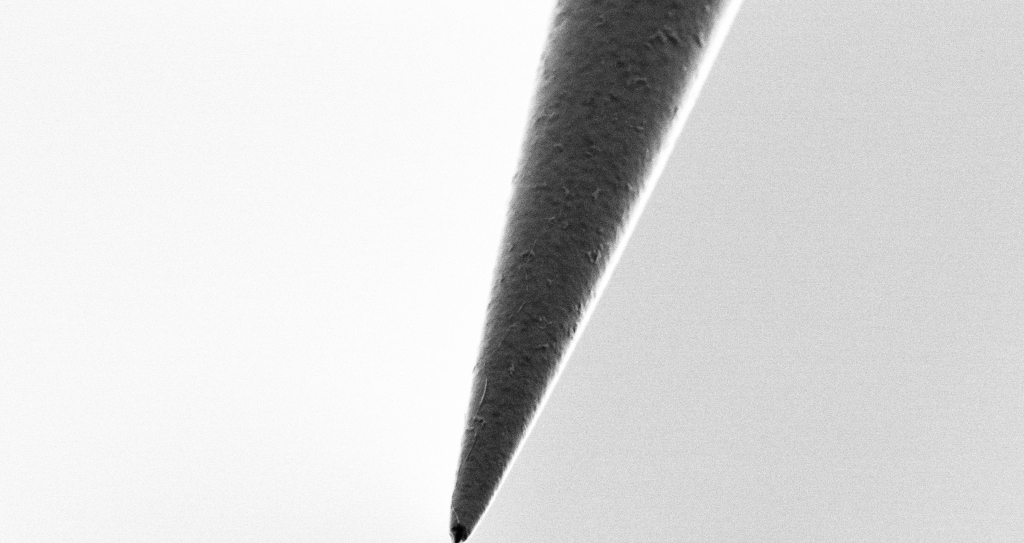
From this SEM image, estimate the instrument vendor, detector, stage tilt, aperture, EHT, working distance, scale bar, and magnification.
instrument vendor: Zeiss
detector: SE2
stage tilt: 45°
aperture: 30 µm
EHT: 1 kV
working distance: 6 mm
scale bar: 2000 nm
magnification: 25 K X